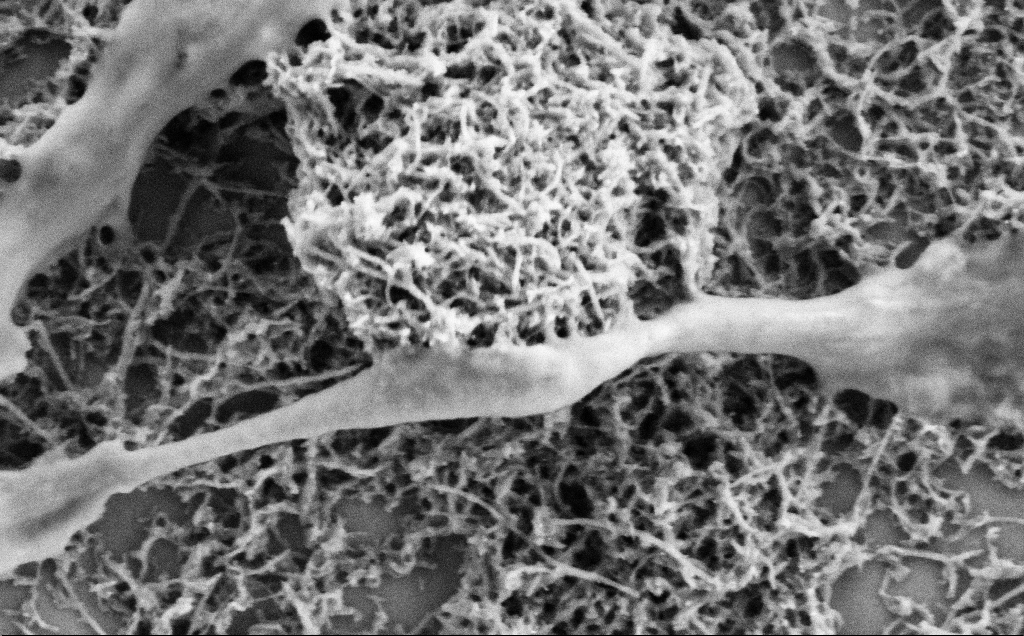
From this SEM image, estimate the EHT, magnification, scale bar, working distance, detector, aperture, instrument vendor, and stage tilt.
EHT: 2 kV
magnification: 60 K X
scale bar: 1000 nm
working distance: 7.1 mm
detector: SE2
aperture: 30 µm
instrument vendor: Zeiss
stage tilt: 0°